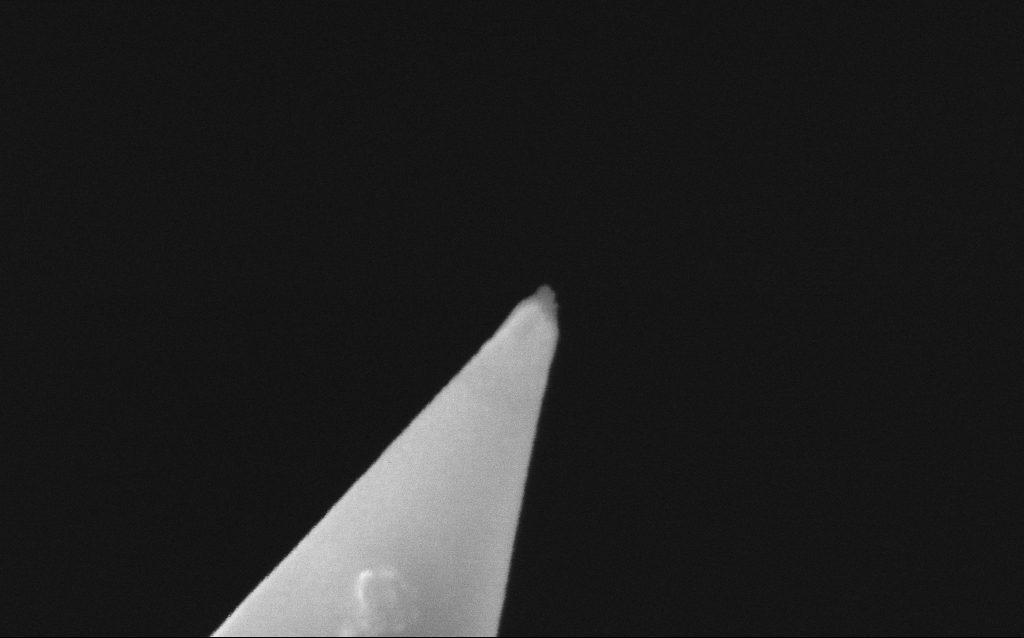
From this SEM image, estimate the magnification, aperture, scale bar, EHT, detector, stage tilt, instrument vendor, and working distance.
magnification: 250 K X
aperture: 30 µm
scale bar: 200 nm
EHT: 5 kV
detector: InLens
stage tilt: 0°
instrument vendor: Zeiss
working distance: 4.7 mm